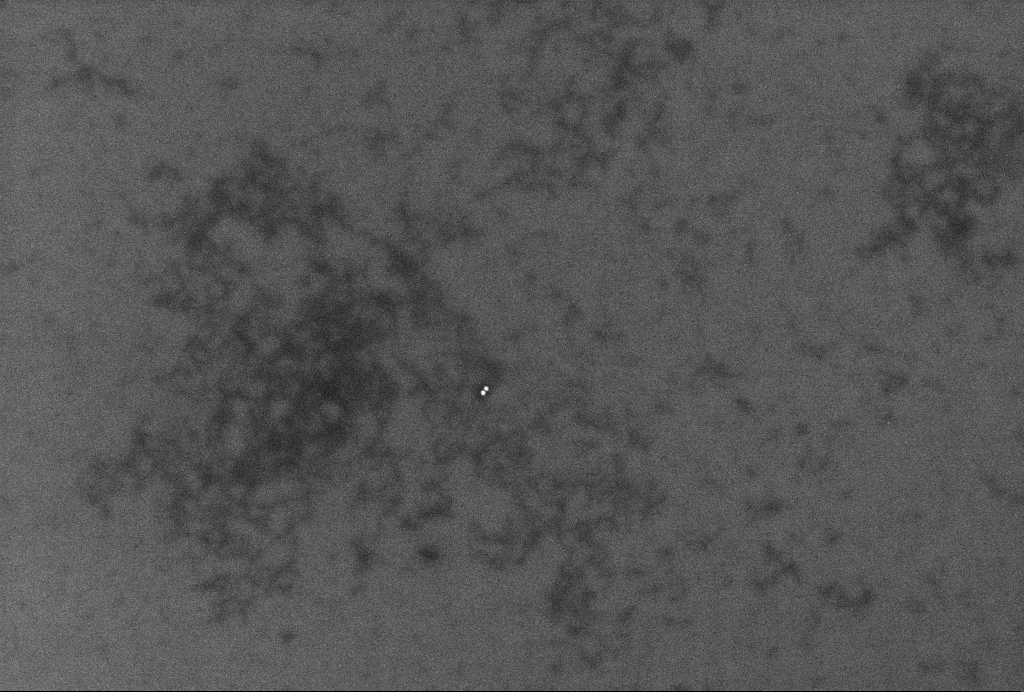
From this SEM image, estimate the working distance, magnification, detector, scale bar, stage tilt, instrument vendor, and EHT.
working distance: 3.3 mm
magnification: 62.65 K X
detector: InLens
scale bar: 200 nm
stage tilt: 0°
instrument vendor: Zeiss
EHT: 2 kV